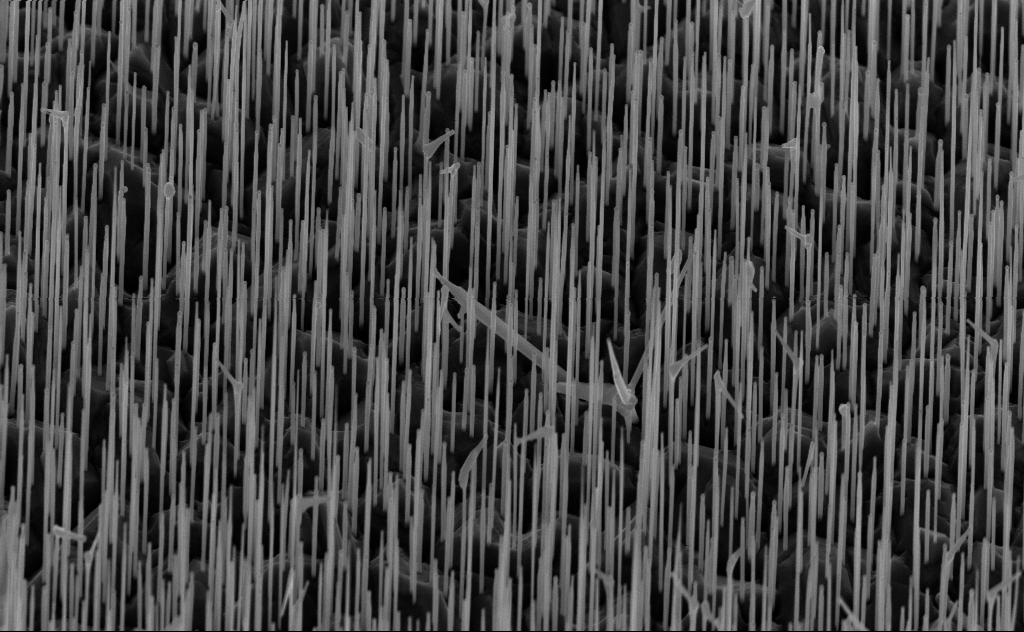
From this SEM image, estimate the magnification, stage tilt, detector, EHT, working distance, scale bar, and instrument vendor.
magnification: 20 K X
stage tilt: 45°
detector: InLens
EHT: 10 kV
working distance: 6 mm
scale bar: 2000 nm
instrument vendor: Zeiss